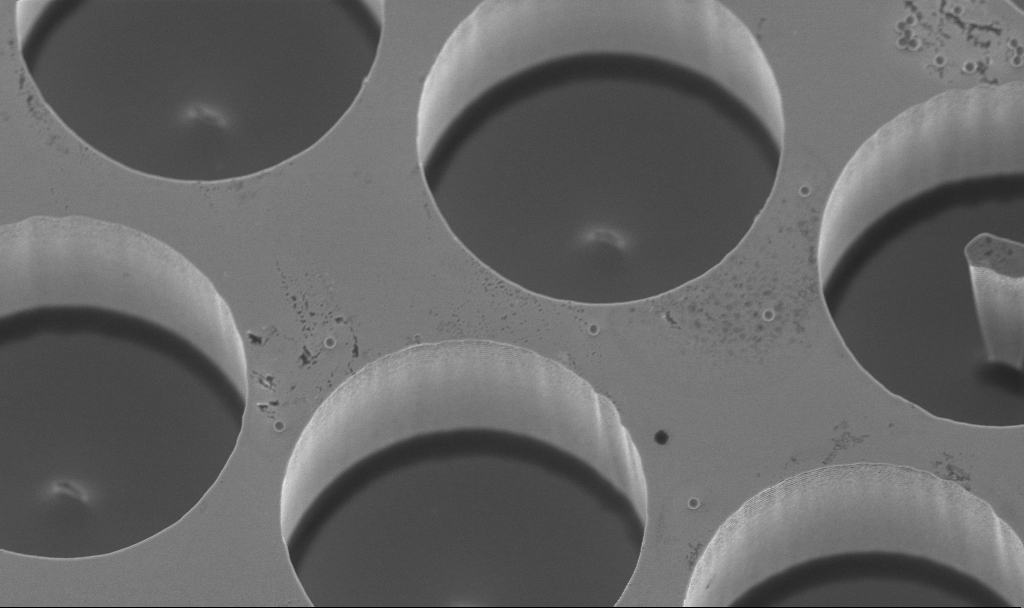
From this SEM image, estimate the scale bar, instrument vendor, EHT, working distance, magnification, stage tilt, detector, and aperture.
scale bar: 10000 nm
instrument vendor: Zeiss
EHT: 3 kV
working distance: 3.1 mm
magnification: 6.76 K X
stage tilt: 20°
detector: InLens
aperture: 30 µm